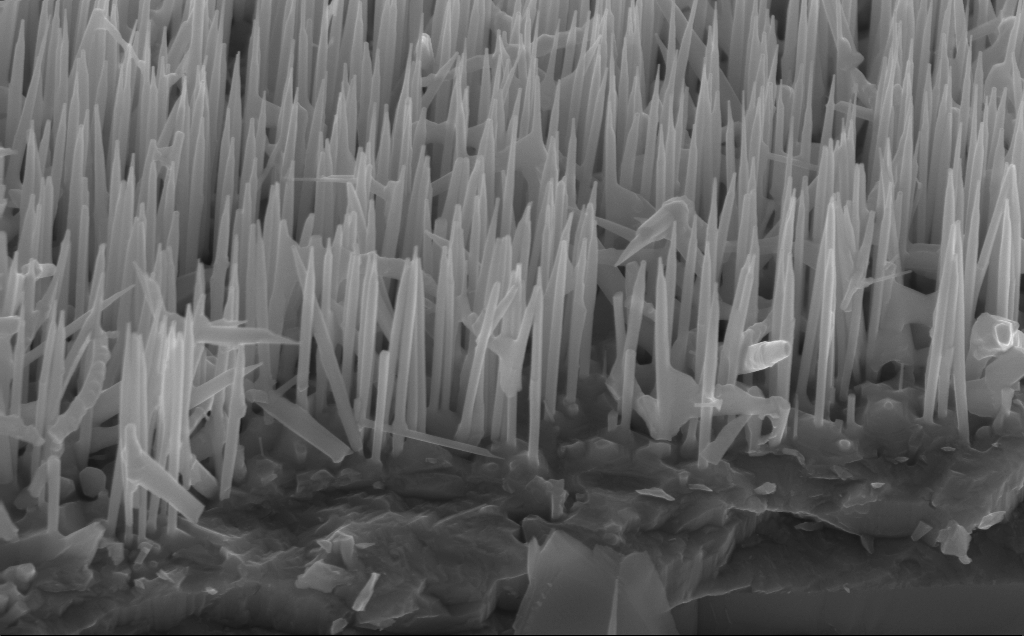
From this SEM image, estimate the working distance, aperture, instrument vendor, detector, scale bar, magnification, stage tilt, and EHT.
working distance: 5 mm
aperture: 30 µm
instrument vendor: Zeiss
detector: InLens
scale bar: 1000 nm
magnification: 40 K X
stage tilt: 45°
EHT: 12 kV